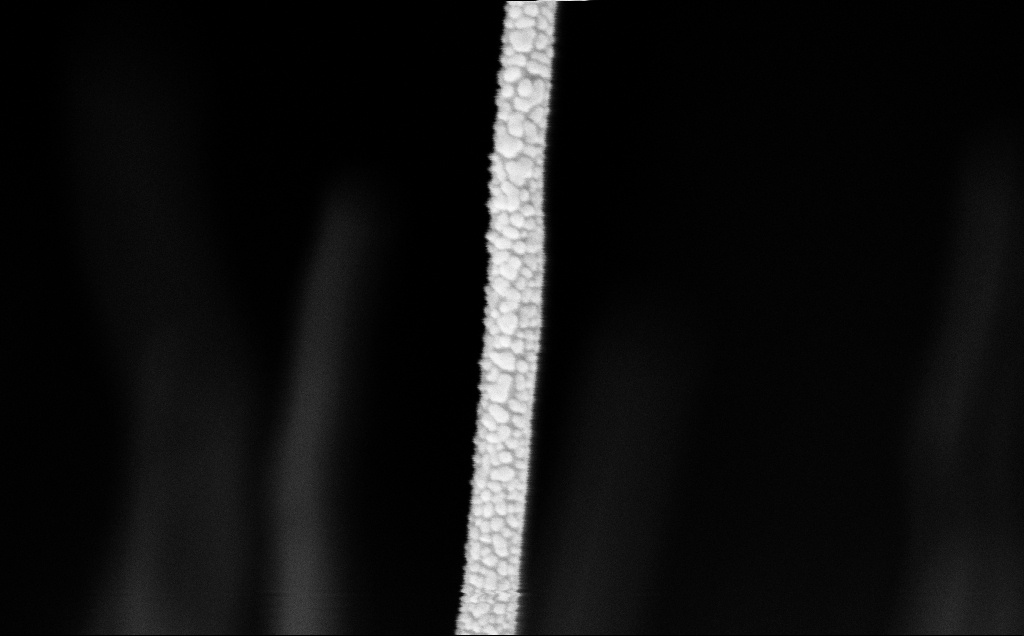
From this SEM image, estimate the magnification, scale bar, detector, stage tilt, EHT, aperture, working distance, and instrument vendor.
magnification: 163.96 K X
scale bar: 200 nm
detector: InLens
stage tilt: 0°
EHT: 5 kV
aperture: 30 µm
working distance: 11 mm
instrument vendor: Zeiss